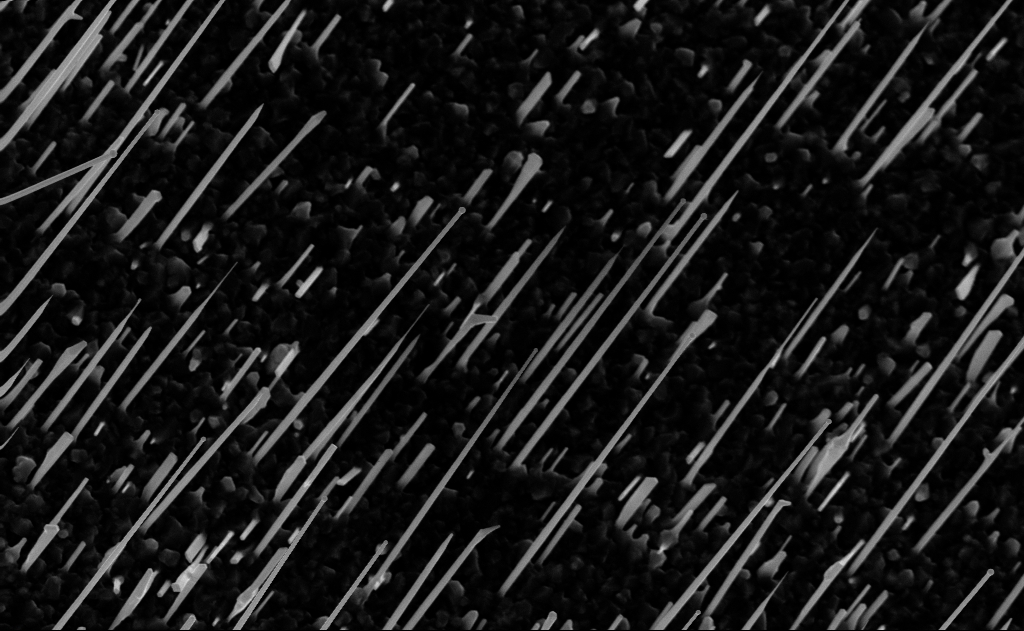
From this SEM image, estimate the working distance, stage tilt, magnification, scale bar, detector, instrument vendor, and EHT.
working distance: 10 mm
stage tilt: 0°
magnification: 20 K X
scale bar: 1000 nm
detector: InLens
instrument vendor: Zeiss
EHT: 10 kV